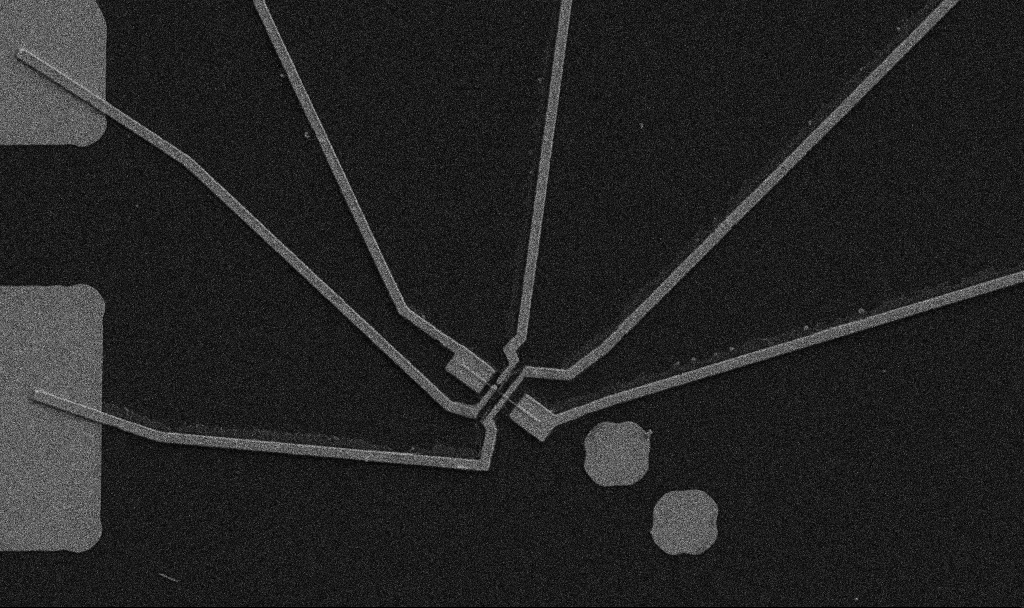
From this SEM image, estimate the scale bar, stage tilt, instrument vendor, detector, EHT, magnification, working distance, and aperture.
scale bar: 10000 nm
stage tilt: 0°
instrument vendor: Zeiss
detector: SE2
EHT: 5 kV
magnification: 5 K X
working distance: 10.7 mm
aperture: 30 µm